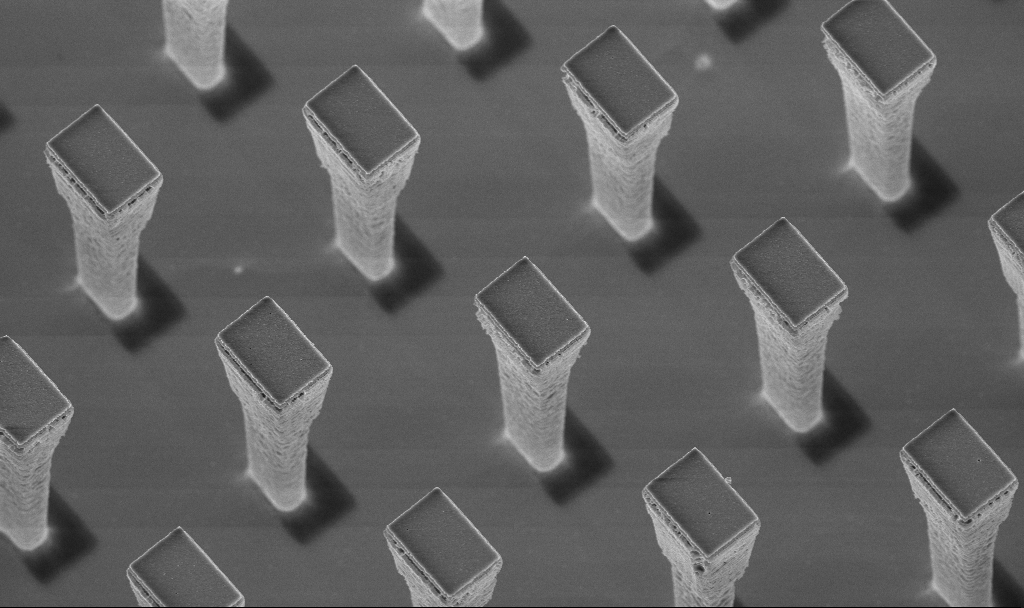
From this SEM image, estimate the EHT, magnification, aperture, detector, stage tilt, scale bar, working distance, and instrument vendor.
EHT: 5 kV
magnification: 8.19 K X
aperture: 30 µm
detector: InLens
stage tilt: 20°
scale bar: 2000 nm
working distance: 4.3 mm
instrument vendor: Zeiss